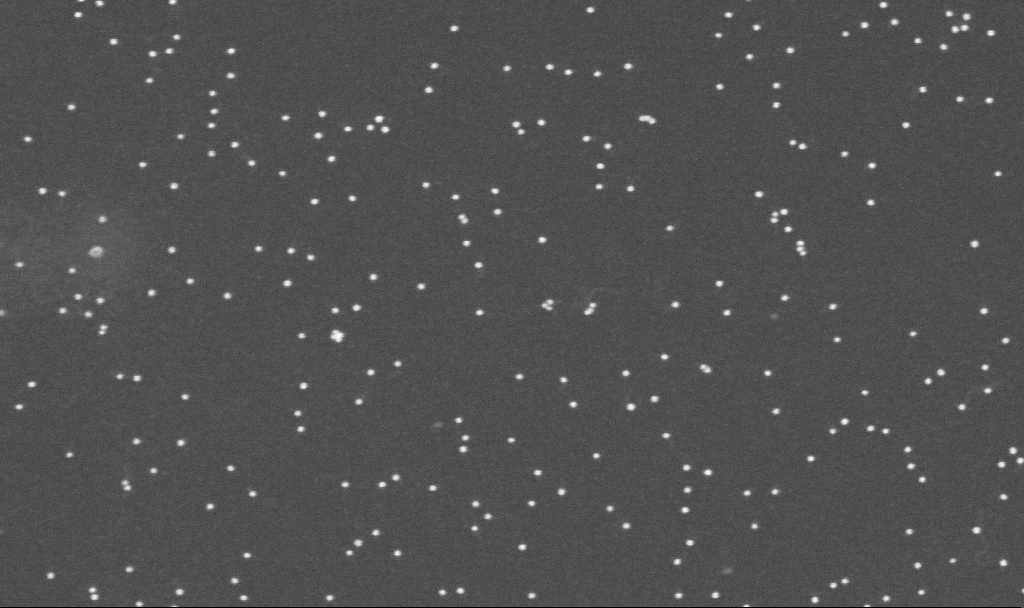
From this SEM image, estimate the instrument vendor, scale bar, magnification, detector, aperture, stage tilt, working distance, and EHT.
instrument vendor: Zeiss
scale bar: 200 nm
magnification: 200 K X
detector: InLens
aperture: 30 µm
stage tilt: -0°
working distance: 3.3 mm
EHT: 10 kV